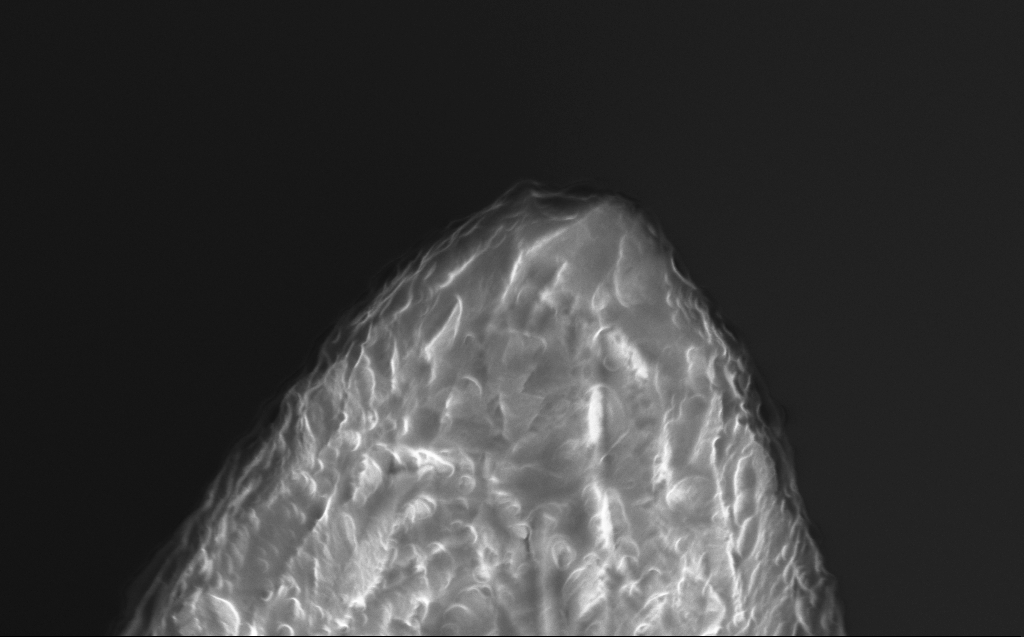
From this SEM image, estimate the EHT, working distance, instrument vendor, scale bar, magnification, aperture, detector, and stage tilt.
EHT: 10 kV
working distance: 5 mm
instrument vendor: Zeiss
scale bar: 1000 nm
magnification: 62.19 K X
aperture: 30 µm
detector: InLens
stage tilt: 40°